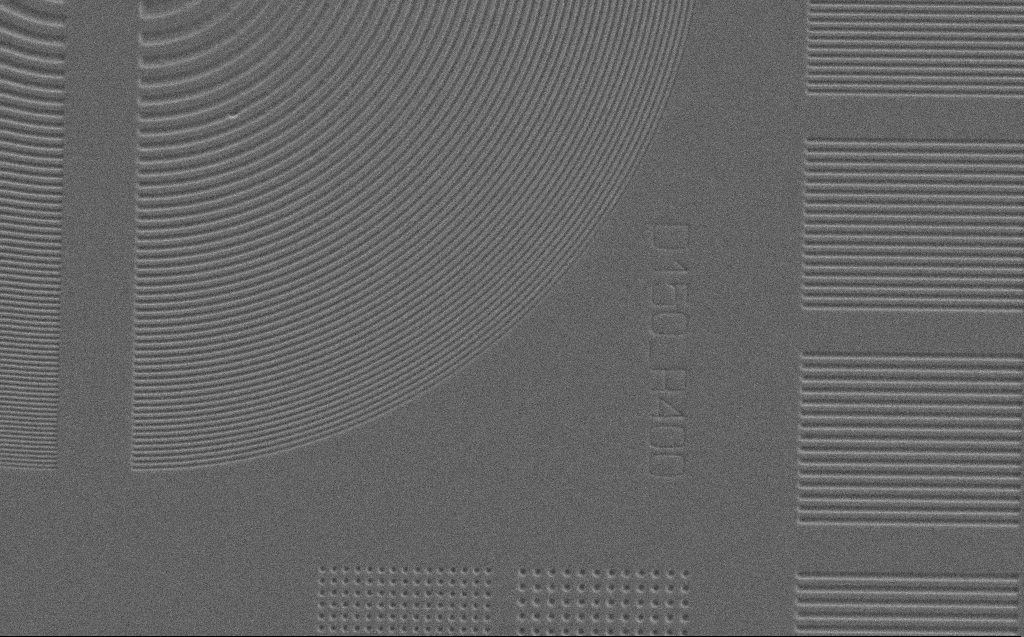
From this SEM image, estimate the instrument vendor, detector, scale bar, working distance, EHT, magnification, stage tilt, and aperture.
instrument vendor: Zeiss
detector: SE2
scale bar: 10000 nm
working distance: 4 mm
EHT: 5 kV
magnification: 2.76 K X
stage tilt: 0°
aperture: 30 µm